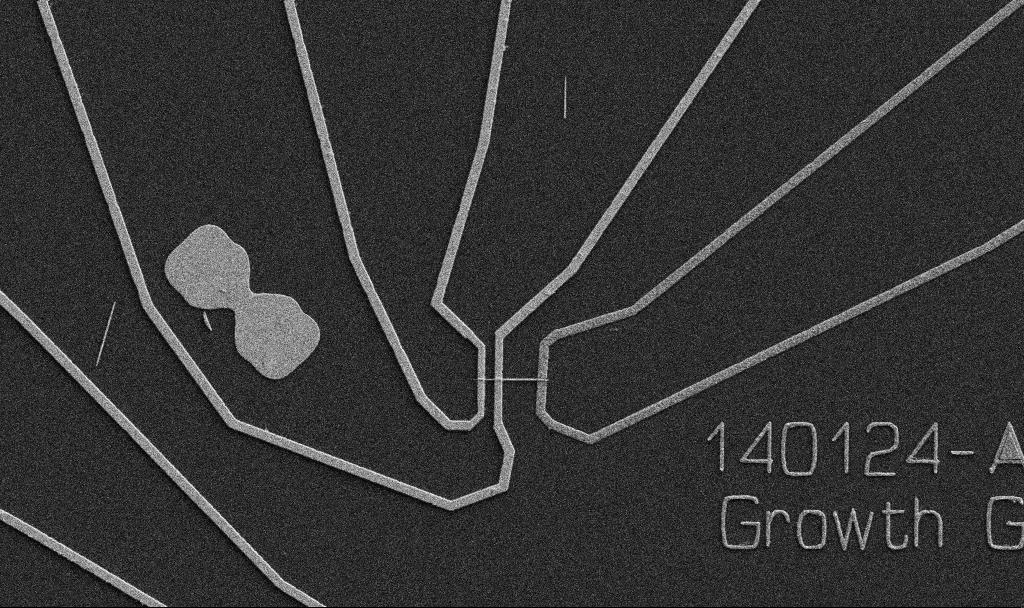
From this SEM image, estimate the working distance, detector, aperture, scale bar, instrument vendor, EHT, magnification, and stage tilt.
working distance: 10.7 mm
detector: SE2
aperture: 30 µm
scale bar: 10000 nm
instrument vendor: Zeiss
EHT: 5 kV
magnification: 5 K X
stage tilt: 0°